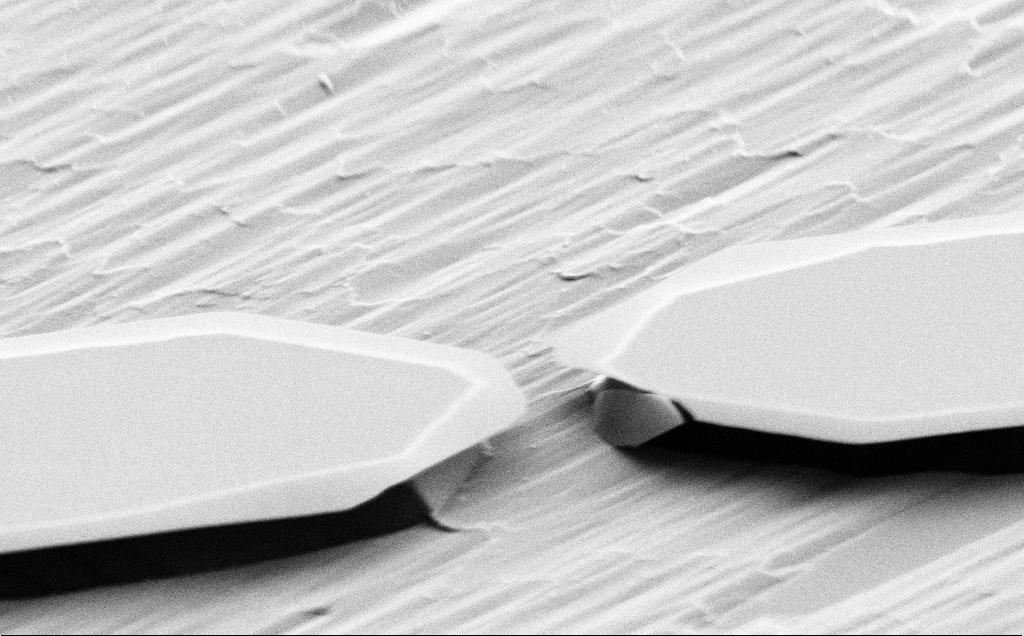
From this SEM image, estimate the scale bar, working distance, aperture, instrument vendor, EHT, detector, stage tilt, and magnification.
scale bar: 2000 nm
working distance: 7 mm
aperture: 30 µm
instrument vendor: Zeiss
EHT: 5 kV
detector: SE2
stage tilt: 70°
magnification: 9.58 K X